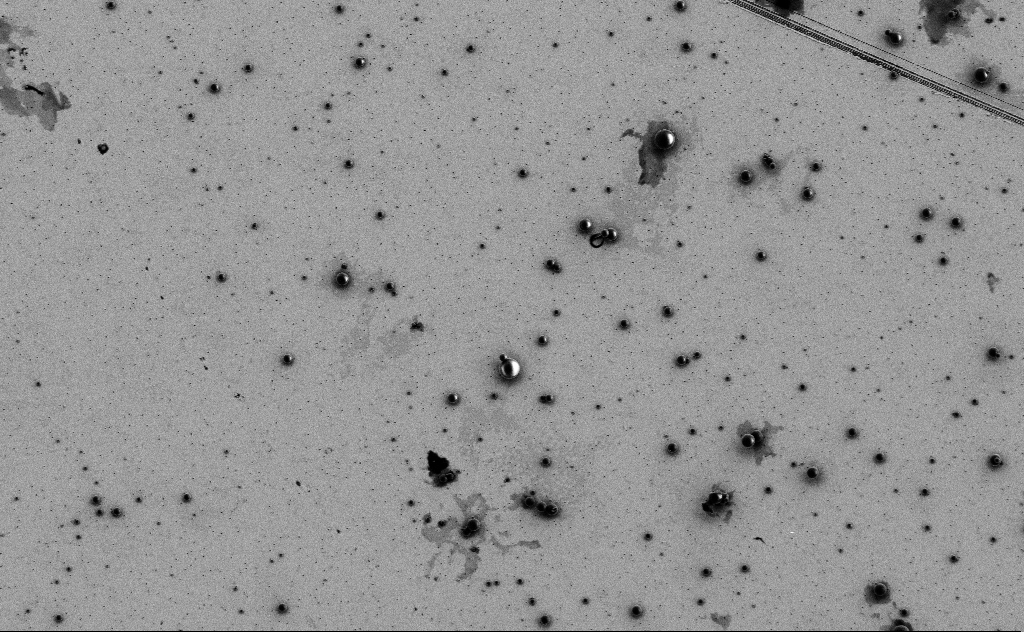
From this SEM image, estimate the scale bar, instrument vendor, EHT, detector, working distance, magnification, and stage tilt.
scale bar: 10000 nm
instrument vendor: Zeiss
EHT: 3 kV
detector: SE2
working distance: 11 mm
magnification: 2.06 K X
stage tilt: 0°